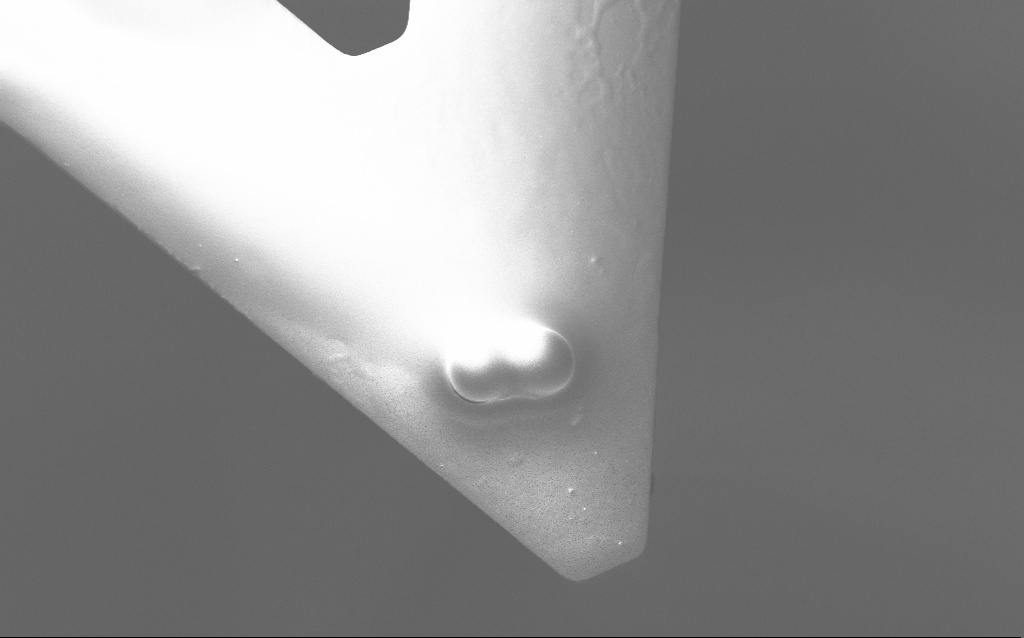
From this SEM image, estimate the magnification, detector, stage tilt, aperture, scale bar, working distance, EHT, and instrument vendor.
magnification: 4.89 K X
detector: InLens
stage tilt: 15.7°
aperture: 30 µm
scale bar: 10000 nm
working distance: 6 mm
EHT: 10 kV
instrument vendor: Zeiss